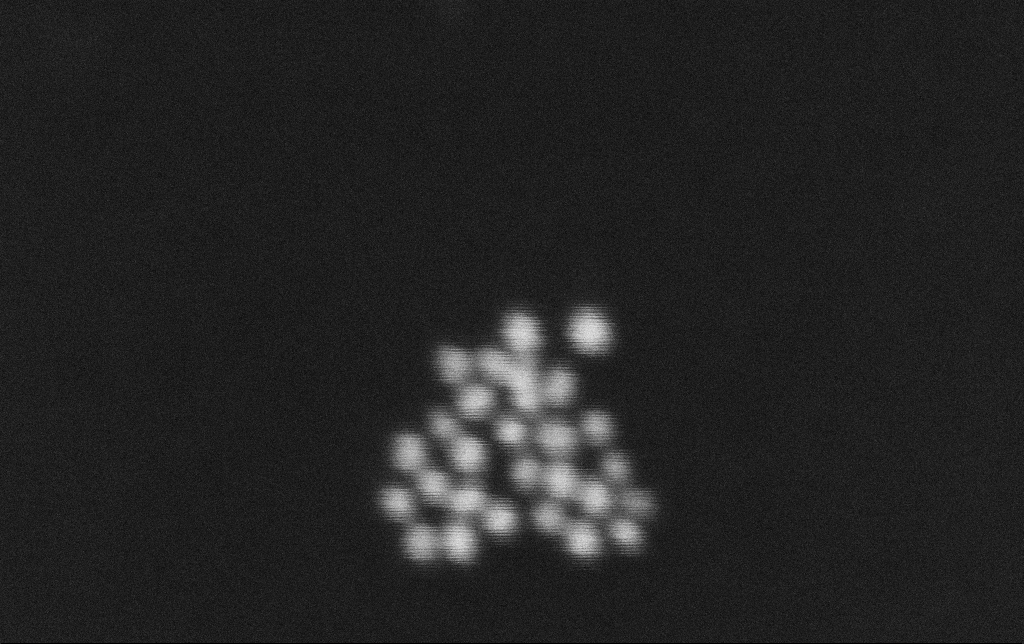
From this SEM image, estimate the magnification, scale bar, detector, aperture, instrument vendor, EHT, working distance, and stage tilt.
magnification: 1000 K X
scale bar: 20 nm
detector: SE2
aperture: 30 µm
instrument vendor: Zeiss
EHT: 30 kV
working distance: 16.8 mm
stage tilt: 0°